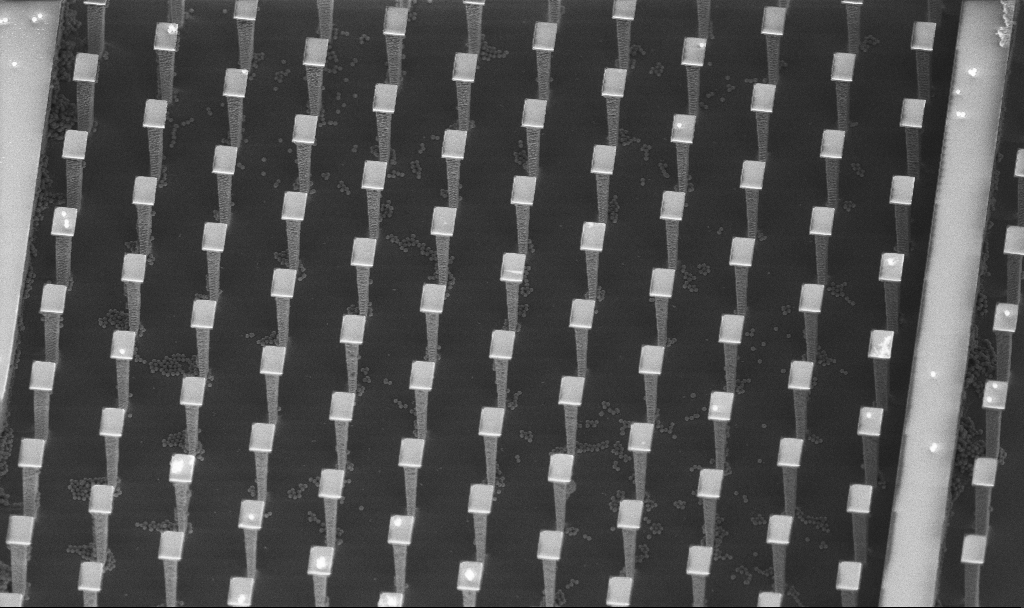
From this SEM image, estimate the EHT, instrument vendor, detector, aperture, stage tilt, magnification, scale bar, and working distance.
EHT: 5 kV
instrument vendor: Zeiss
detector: InLens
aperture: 30 µm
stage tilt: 30°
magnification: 2.75 K X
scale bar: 10000 nm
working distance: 5.1 mm